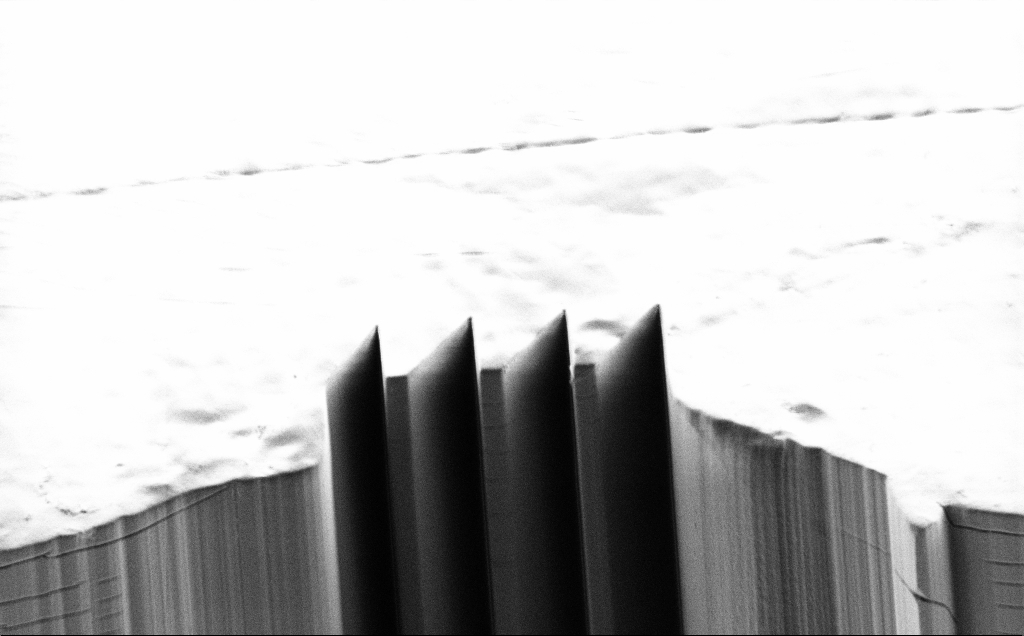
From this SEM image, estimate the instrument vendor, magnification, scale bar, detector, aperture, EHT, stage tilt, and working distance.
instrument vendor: Zeiss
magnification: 2.98 K X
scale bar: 20000 nm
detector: SE2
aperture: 30 µm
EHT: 1.2 kV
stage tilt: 45°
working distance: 7 mm